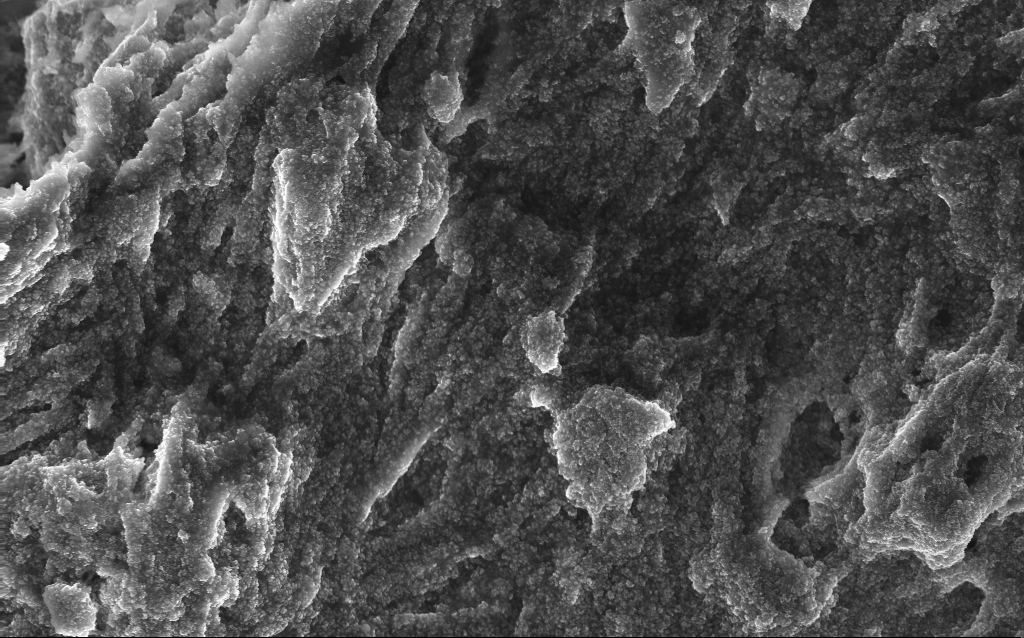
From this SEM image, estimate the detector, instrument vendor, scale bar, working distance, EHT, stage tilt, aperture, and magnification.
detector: InLens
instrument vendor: Zeiss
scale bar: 1000 nm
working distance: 2.6 mm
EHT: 10 kV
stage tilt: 0°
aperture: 30 µm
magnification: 15.33 K X